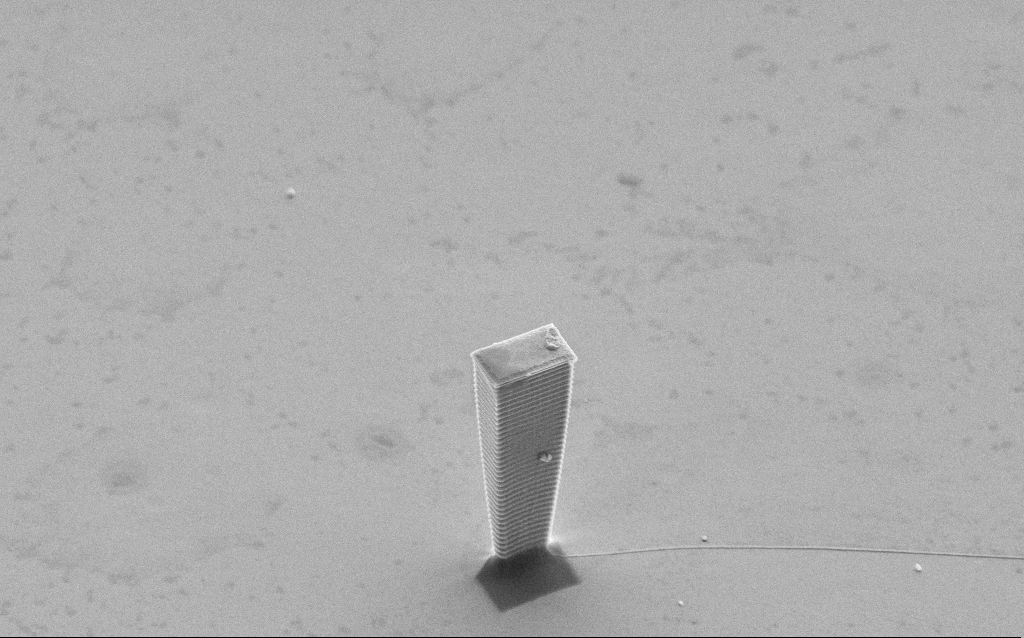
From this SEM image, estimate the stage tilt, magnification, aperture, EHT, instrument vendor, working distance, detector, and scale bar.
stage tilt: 45°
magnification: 6.96 K X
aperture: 30 µm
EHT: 5 kV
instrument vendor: Zeiss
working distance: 12 mm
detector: SE2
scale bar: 10000 nm